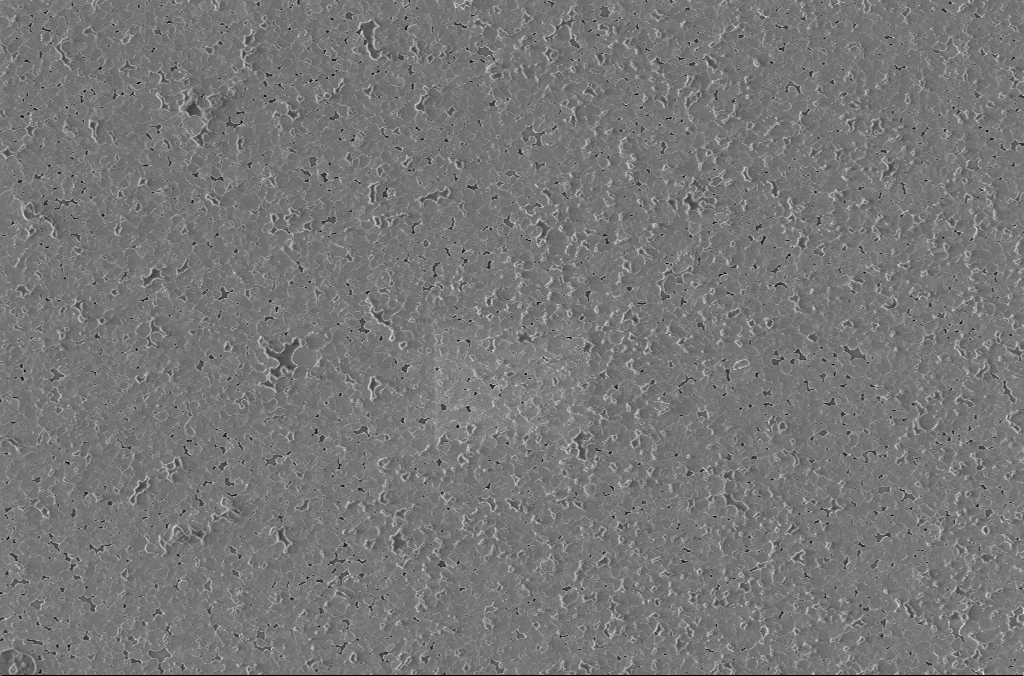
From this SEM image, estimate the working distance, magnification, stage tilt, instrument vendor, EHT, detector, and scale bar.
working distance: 3 mm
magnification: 5 K X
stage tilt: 0°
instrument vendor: Zeiss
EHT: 2 kV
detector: InLens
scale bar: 10000 nm